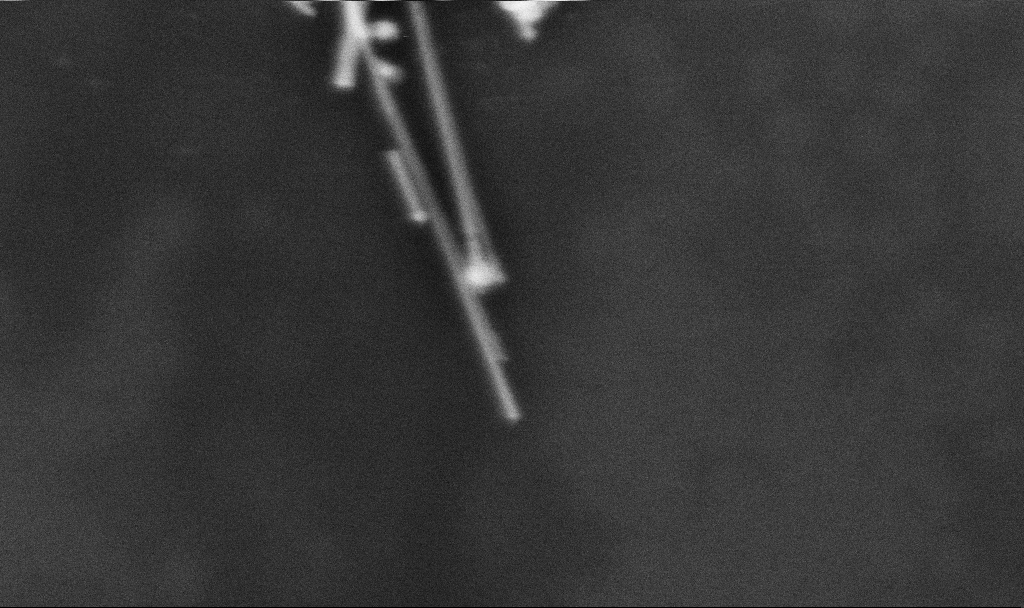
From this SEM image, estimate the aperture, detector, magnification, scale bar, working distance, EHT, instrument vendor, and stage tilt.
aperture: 30 µm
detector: InLens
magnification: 155.11 K X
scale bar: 200 nm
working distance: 3.3 mm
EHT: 3 kV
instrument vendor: Zeiss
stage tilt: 0°